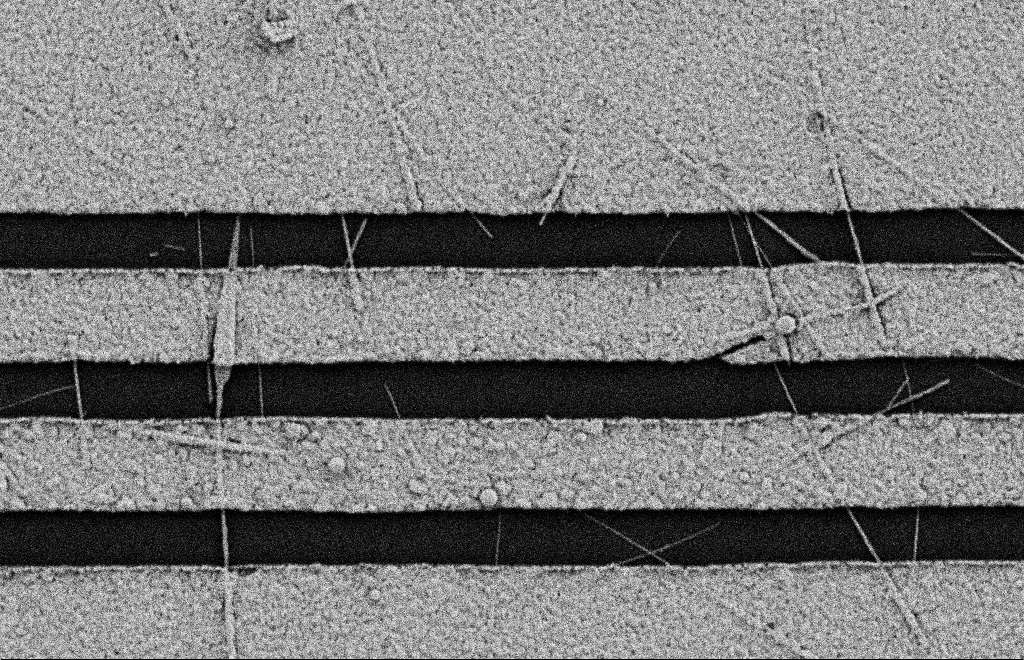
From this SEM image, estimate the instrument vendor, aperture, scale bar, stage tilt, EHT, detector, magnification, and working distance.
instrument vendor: Zeiss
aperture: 20 µm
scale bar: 2000 nm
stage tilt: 0°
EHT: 2 kV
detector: SE2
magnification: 13.69 K X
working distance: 11 mm